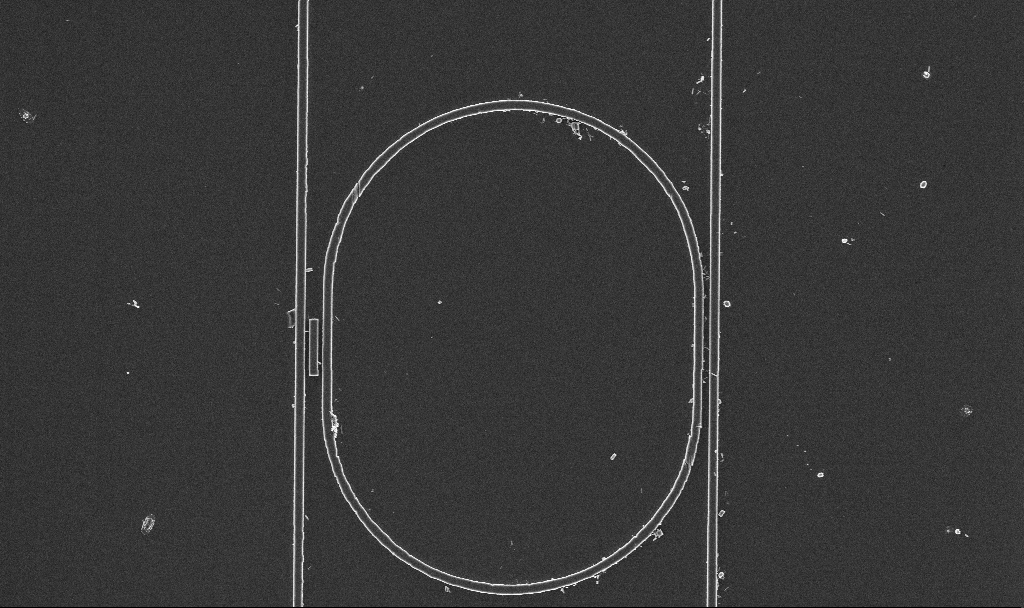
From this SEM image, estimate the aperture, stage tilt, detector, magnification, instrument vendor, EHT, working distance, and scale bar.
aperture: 30 µm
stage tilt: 0°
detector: InLens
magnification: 7.1 K X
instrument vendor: Zeiss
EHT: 3 kV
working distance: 2.9 mm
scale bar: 10000 nm